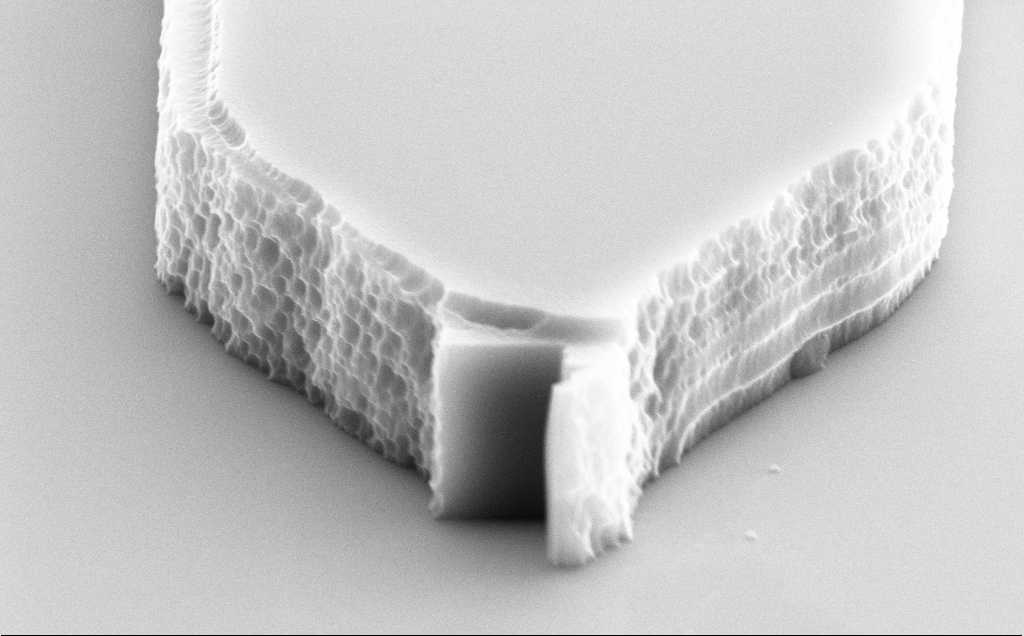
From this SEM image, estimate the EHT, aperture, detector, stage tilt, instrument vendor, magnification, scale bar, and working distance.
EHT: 10 kV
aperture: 30 µm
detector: SE2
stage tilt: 70°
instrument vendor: Zeiss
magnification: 30.82 K X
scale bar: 1000 nm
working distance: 9 mm